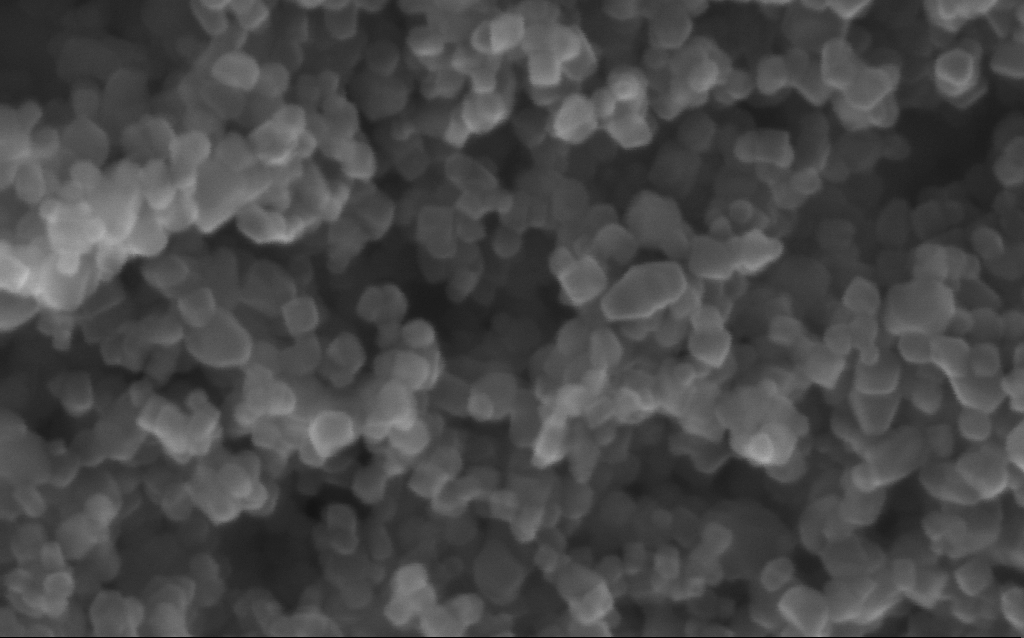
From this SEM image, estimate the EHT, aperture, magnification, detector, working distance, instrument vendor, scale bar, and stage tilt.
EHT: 10 kV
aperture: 30 µm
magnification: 600 K X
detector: InLens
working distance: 2.7 mm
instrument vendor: Zeiss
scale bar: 100 nm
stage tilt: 0°